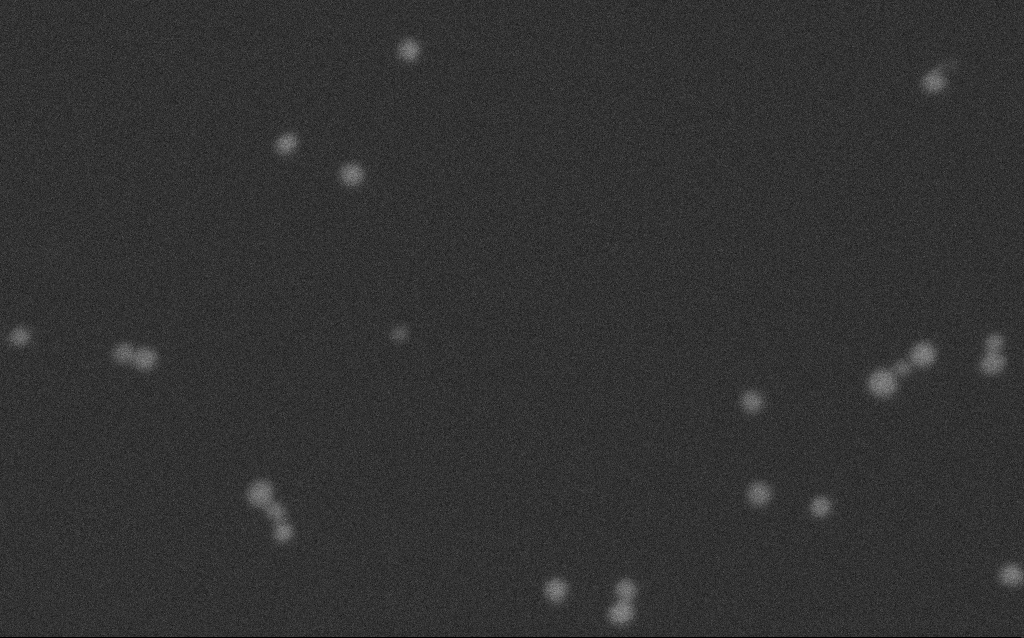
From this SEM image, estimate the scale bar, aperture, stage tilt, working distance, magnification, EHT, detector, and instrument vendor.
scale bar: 100 nm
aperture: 30 µm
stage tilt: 0°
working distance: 2.7 mm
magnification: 657.61 K X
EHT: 8 kV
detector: SE2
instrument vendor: Zeiss